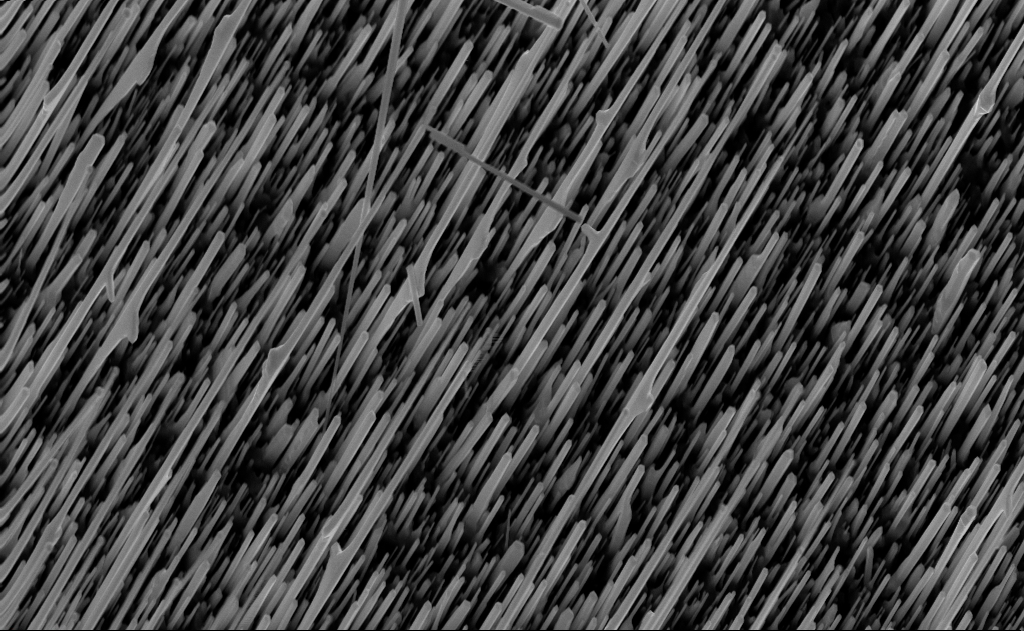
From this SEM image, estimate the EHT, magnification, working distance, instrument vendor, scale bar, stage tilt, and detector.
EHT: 10 kV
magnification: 20 K X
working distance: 5 mm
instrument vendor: Zeiss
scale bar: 2000 nm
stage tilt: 0°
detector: InLens